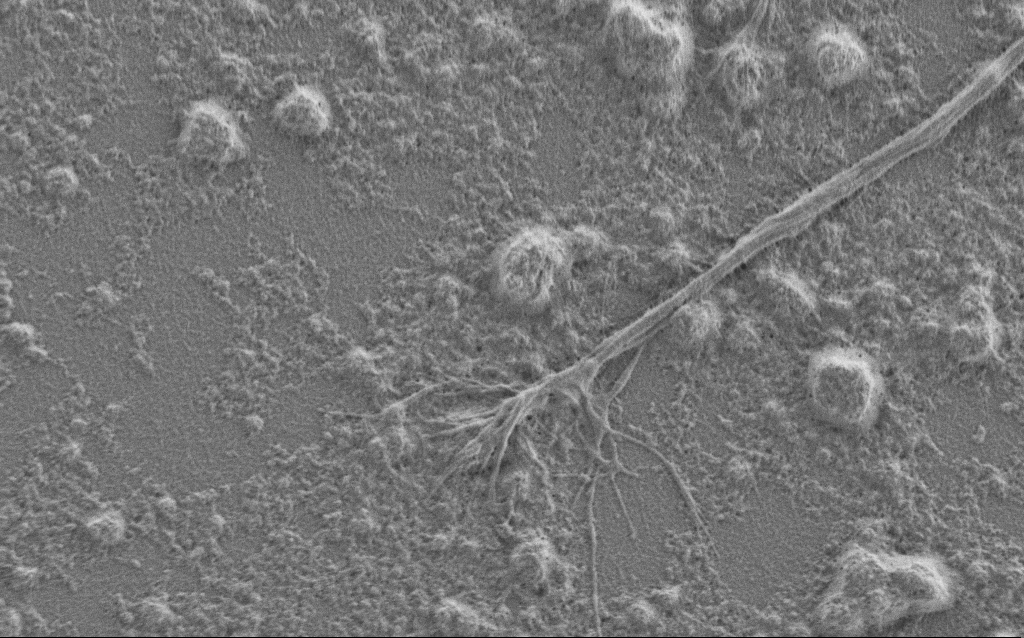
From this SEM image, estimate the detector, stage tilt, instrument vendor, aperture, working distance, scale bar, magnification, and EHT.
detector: SE2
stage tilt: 0°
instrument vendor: Zeiss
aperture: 30 µm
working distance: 6 mm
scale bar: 2000 nm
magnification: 7.5 K X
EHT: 1 kV